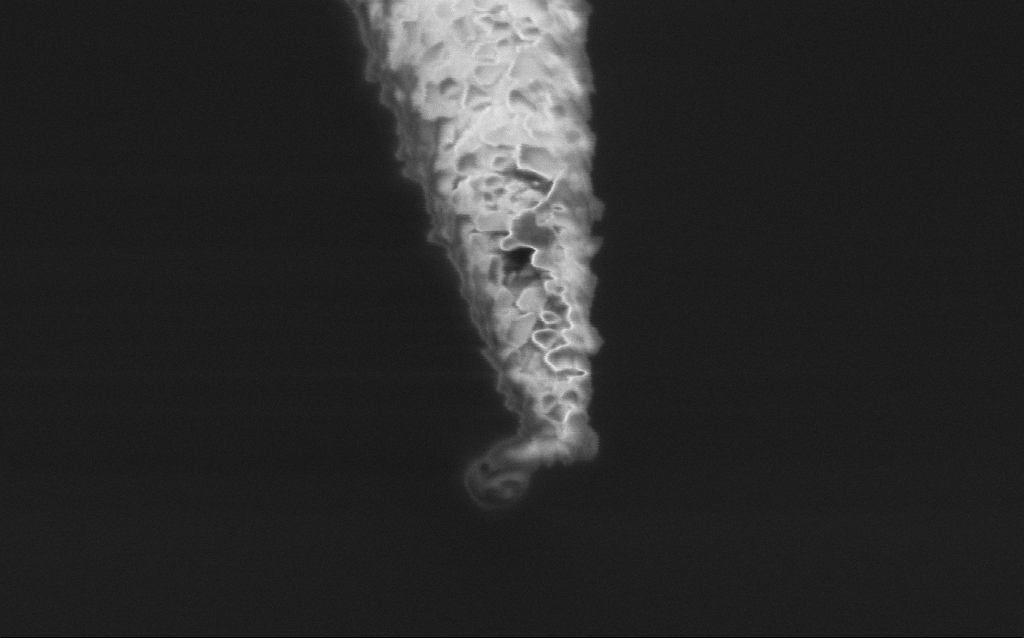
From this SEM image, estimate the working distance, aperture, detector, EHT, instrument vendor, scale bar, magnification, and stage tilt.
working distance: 7.6 mm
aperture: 30 µm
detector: InLens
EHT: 2 kV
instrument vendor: Zeiss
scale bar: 100 nm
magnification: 150 K X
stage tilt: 45°